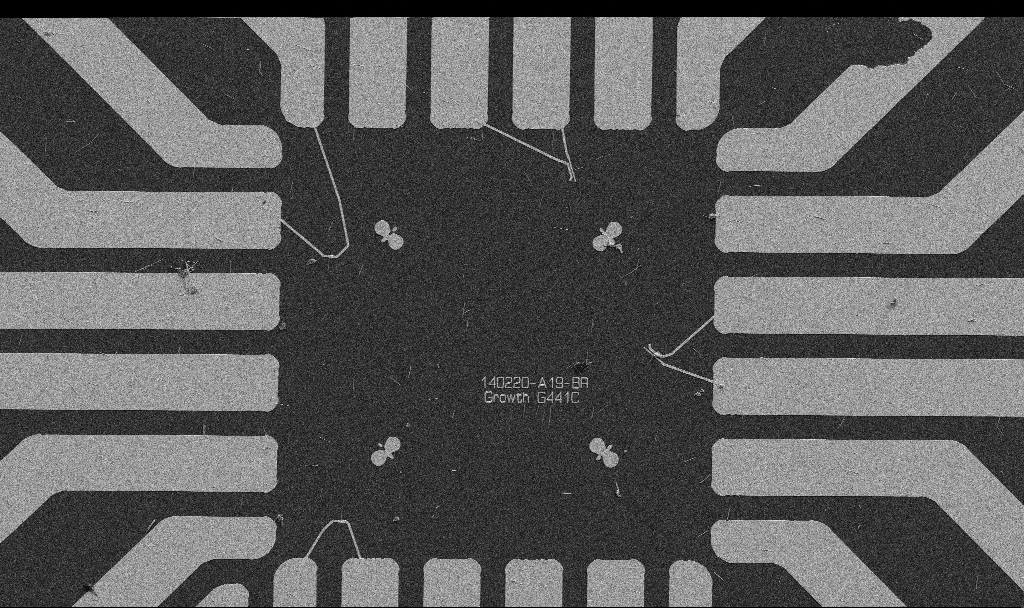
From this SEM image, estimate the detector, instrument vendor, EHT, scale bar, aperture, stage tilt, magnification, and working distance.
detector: SE2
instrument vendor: Zeiss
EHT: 5 kV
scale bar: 20000 nm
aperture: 30 µm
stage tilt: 0°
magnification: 1 K X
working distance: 10.7 mm